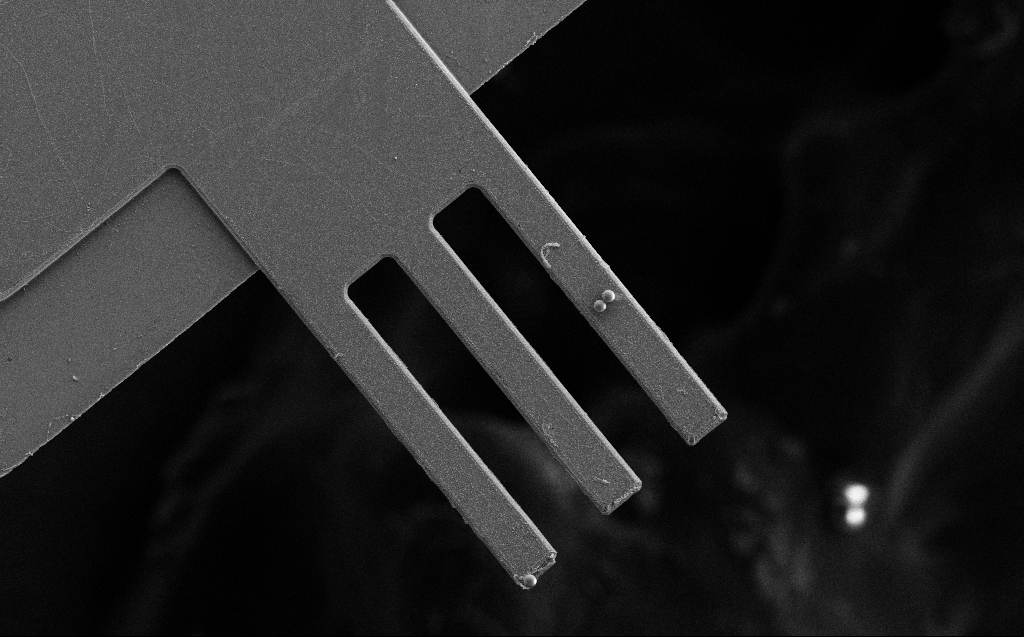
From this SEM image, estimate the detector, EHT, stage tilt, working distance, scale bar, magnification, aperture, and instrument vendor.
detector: SE2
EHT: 10 kV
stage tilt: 0°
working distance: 5 mm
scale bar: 20000 nm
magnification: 1.02 K X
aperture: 30 µm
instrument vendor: Zeiss